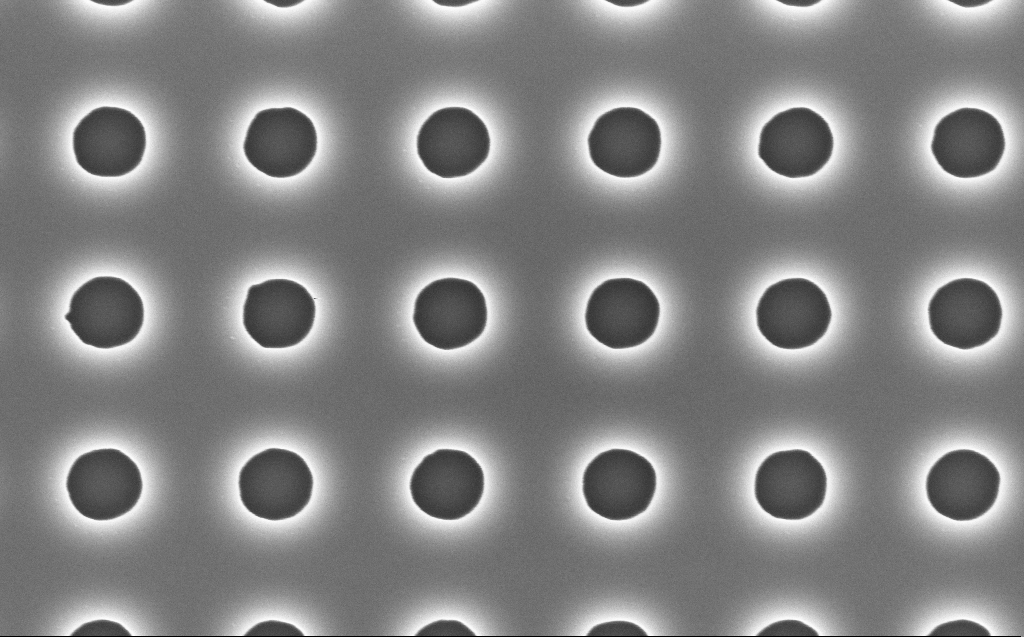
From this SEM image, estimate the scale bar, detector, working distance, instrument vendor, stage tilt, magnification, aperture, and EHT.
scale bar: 1000 nm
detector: InLens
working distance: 7 mm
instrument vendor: Zeiss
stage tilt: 0°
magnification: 60 K X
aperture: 30 µm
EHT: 5 kV